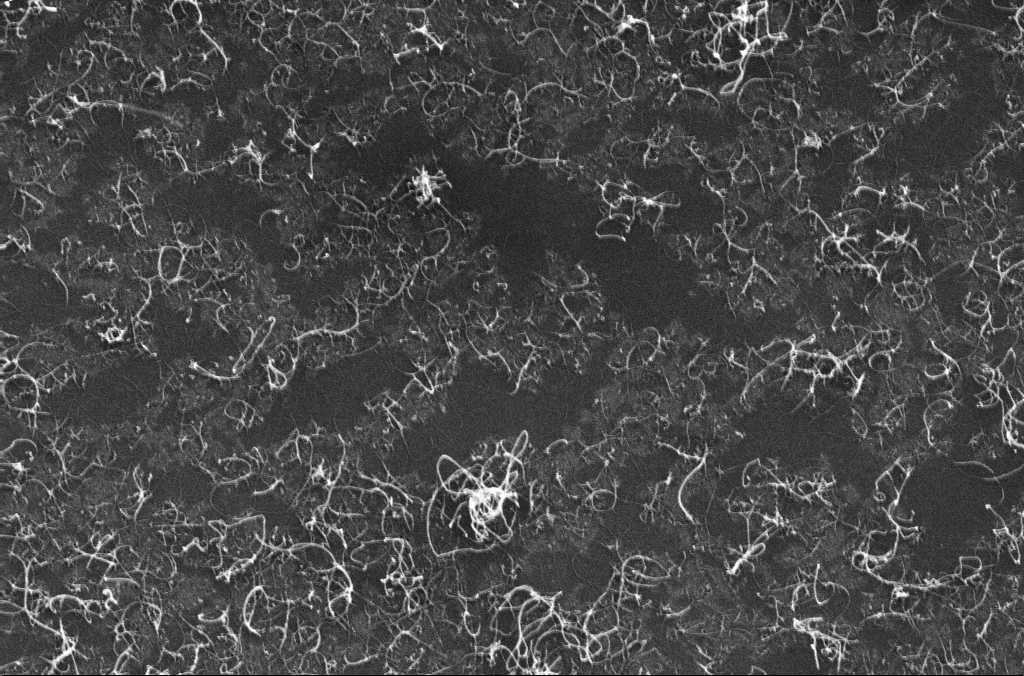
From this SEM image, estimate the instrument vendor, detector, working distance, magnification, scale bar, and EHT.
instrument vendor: Zeiss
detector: InLens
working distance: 3.2 mm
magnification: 71.6 K X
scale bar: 200 nm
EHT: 10 kV